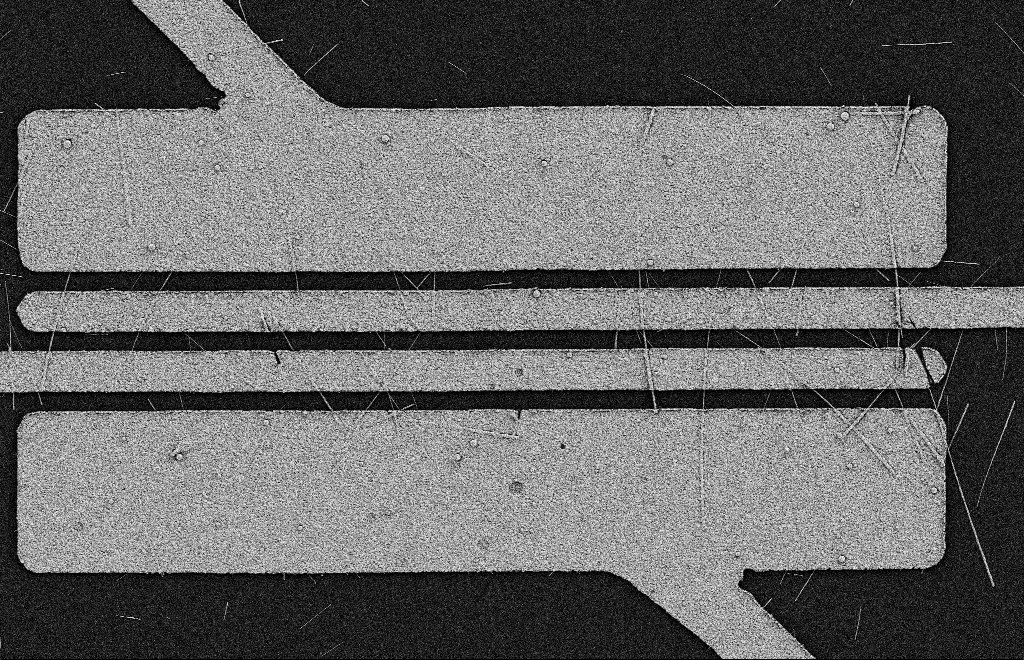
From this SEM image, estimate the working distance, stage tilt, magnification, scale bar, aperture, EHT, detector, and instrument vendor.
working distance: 11 mm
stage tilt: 0°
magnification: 5.52 K X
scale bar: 2000 nm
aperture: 20 µm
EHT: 2 kV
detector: SE2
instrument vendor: Zeiss